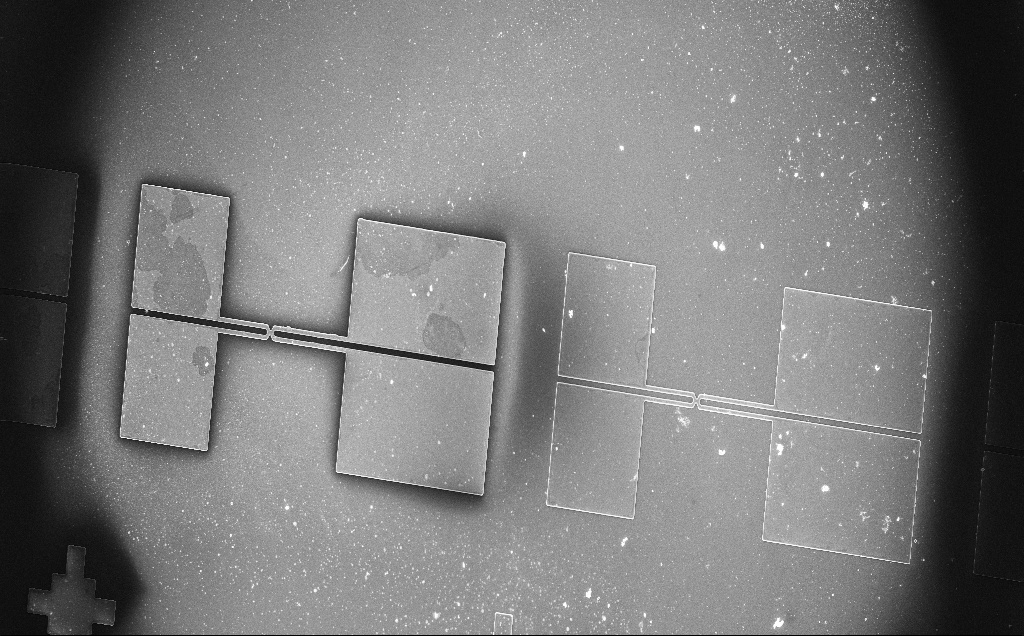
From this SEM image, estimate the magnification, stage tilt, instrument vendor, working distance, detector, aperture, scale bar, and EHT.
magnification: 0.16 K X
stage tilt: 0°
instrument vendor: Zeiss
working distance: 4 mm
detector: InLens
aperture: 30 µm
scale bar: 100000 nm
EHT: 15 kV